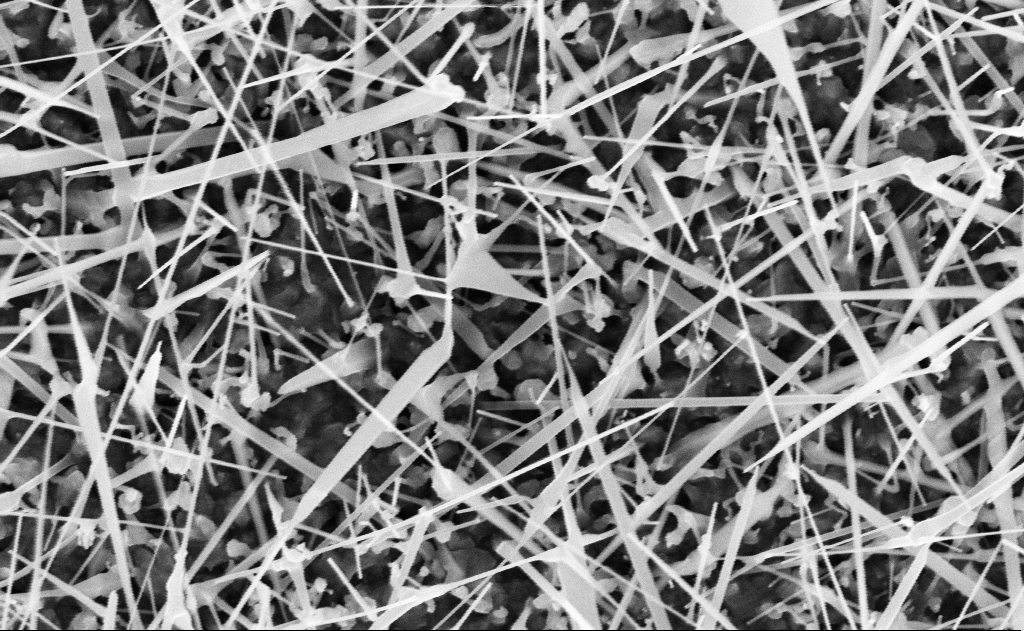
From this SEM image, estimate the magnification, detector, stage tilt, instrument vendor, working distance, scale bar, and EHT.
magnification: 40 K X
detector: InLens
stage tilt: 0°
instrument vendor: Zeiss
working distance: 20 mm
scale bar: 1000 nm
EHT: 10 kV